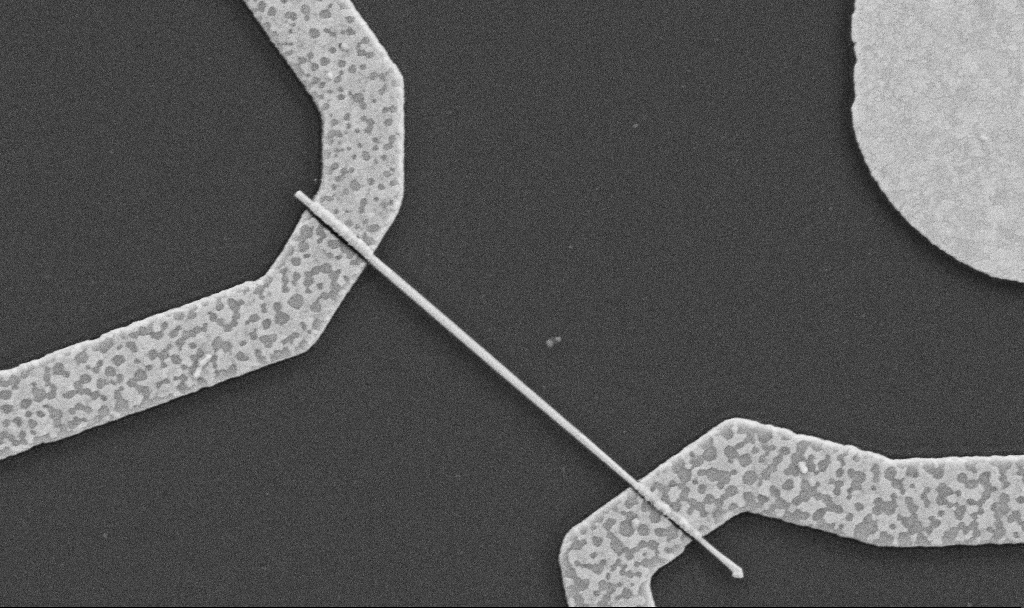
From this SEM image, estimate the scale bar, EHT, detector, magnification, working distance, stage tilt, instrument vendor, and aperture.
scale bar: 1000 nm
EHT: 5 kV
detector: SE2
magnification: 30 K X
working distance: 8.7 mm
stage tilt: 0°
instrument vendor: Zeiss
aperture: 30 µm